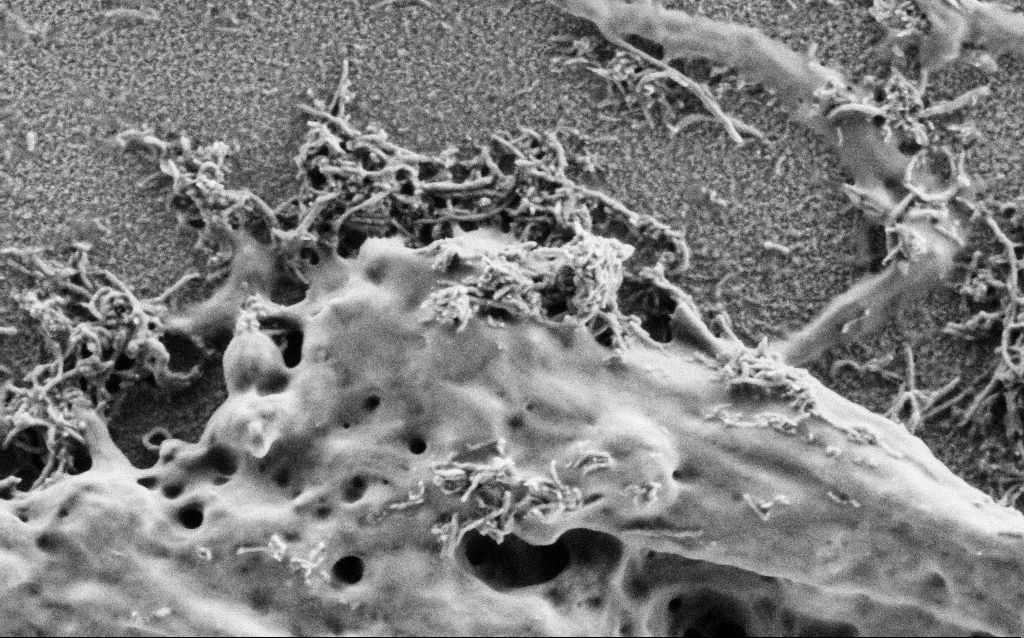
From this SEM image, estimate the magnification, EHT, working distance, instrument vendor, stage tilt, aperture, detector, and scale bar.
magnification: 75 K X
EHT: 1 kV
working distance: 4 mm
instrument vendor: Zeiss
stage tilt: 0°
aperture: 30 µm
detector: SE2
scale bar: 200 nm